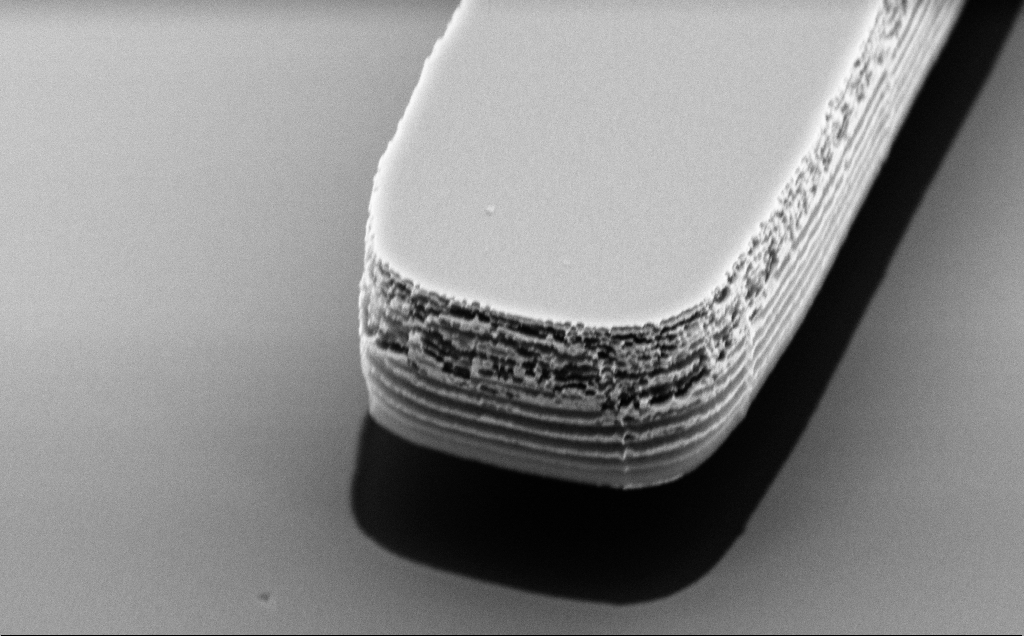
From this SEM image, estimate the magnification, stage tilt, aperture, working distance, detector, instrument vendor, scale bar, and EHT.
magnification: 32.58 K X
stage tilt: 50°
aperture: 30 µm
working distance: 10 mm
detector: SE2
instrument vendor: Zeiss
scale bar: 2000 nm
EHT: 5 kV